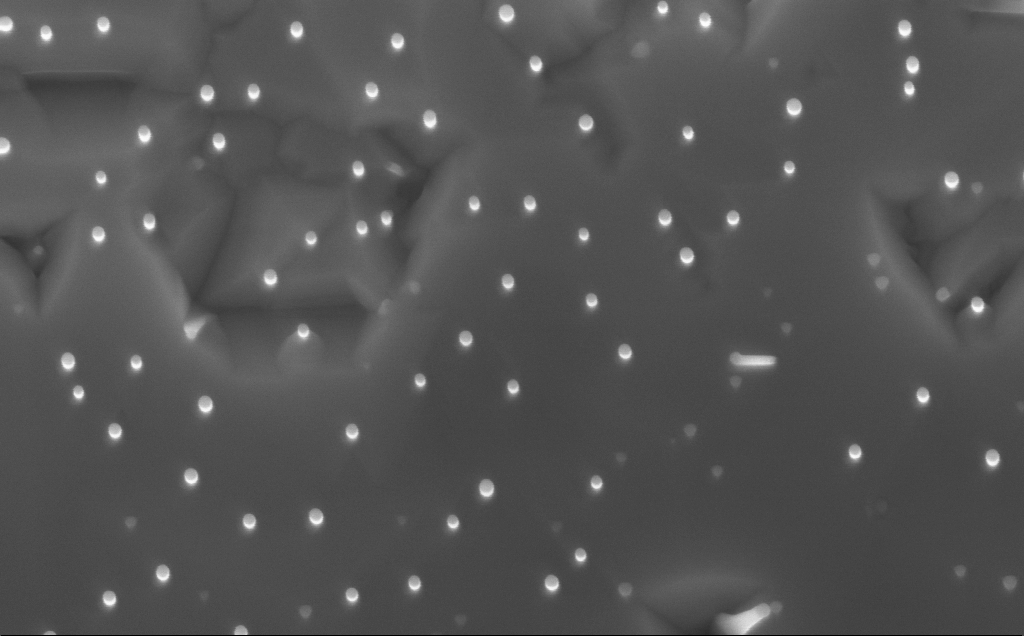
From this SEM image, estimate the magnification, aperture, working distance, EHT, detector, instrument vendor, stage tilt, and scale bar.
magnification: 100 K X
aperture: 30 µm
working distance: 5 mm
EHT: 10 kV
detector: InLens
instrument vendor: Zeiss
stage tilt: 0°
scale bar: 200 nm